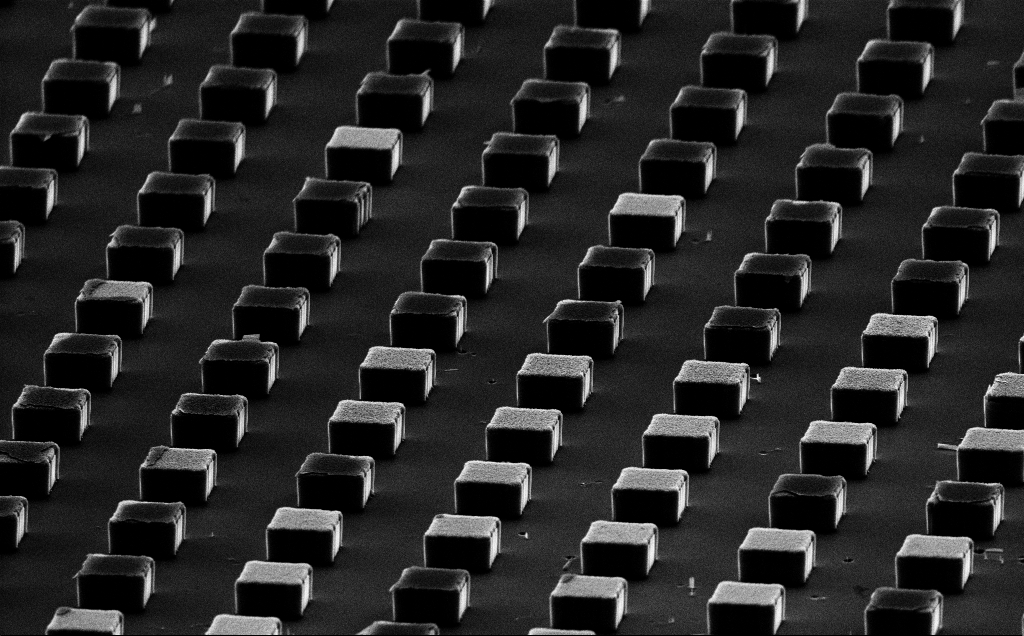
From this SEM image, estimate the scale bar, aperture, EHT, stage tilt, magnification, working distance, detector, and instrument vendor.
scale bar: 10000 nm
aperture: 30 µm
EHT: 10 kV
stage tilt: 70°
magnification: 2.93 K X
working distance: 18 mm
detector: SE2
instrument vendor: Zeiss